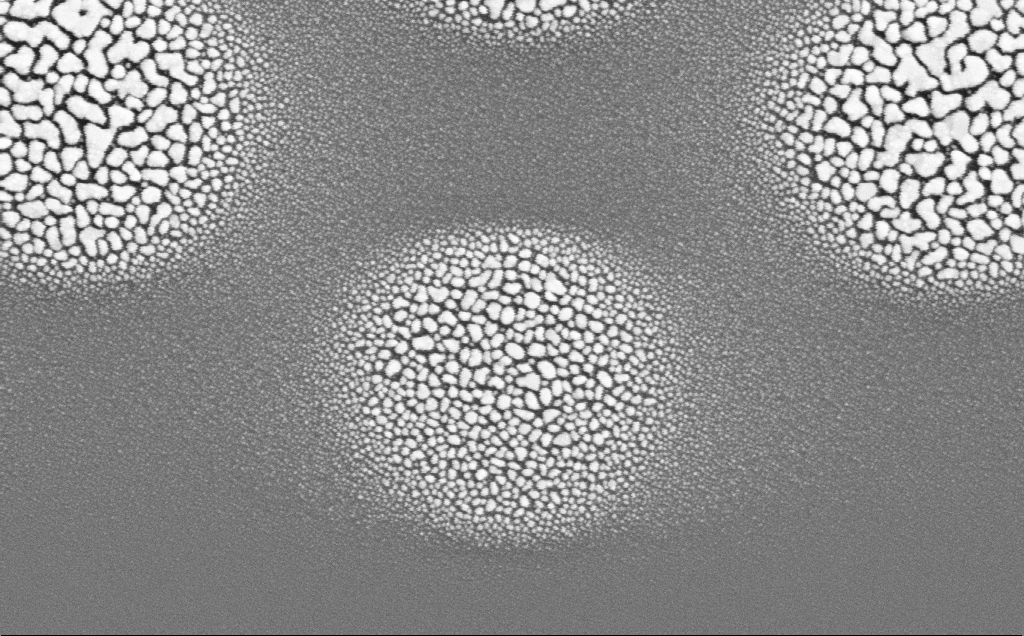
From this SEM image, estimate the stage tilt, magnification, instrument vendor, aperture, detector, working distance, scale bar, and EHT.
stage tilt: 0°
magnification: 60.72 K X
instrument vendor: Zeiss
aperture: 30 µm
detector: SE2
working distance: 20 mm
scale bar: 1000 nm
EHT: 5 kV